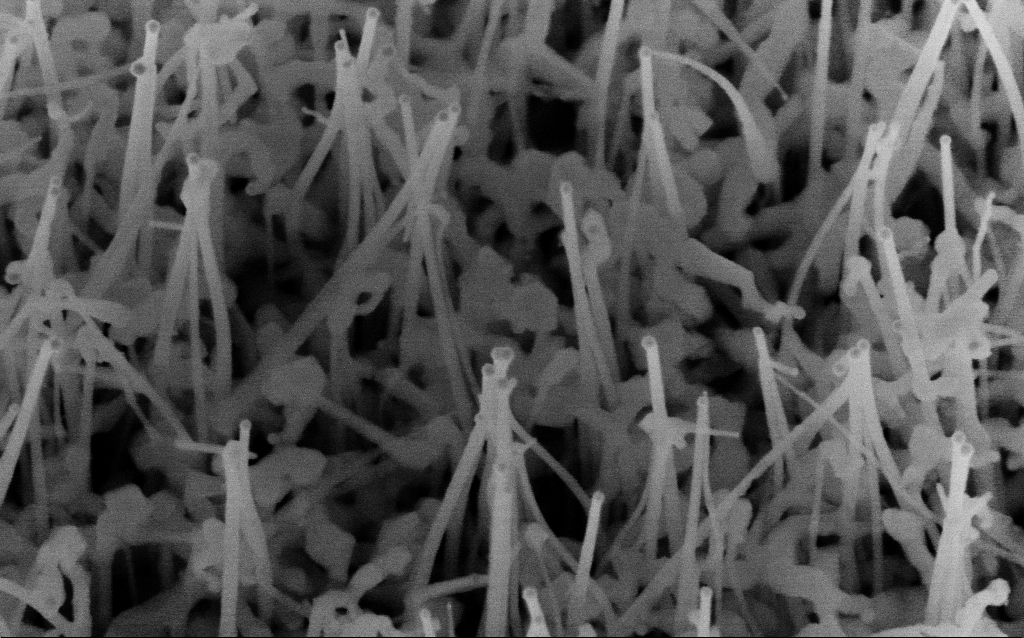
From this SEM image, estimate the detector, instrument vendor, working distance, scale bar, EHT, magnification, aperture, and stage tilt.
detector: InLens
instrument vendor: Zeiss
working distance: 7.3 mm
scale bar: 100 nm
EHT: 10 kV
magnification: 149.8 K X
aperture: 30 µm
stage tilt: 35°